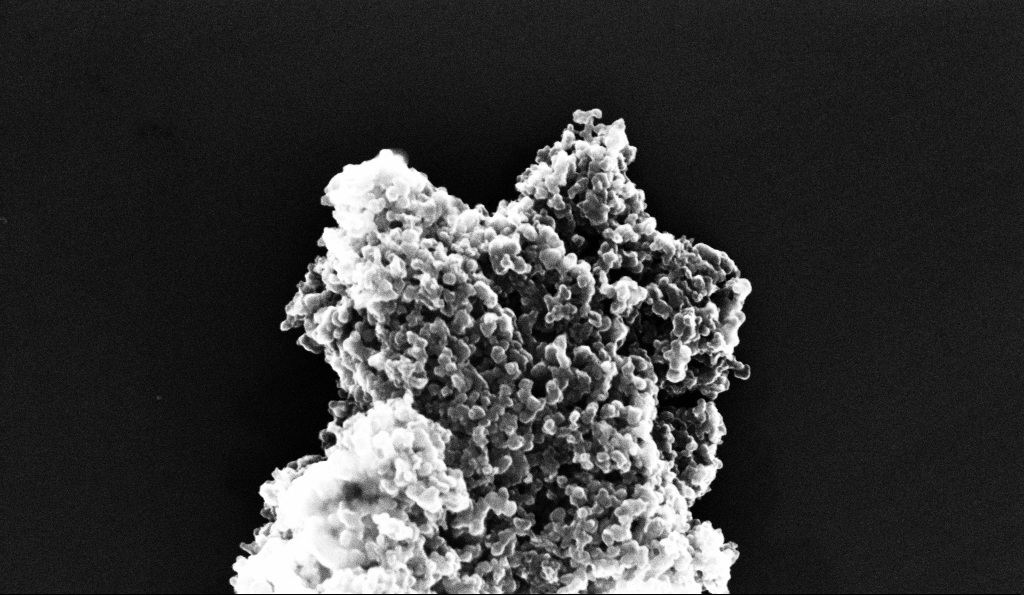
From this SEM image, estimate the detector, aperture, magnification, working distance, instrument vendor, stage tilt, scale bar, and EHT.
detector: InLens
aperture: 30 µm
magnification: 107.88 K X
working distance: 5.3 mm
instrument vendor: Zeiss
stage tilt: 0°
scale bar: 200 nm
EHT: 10 kV